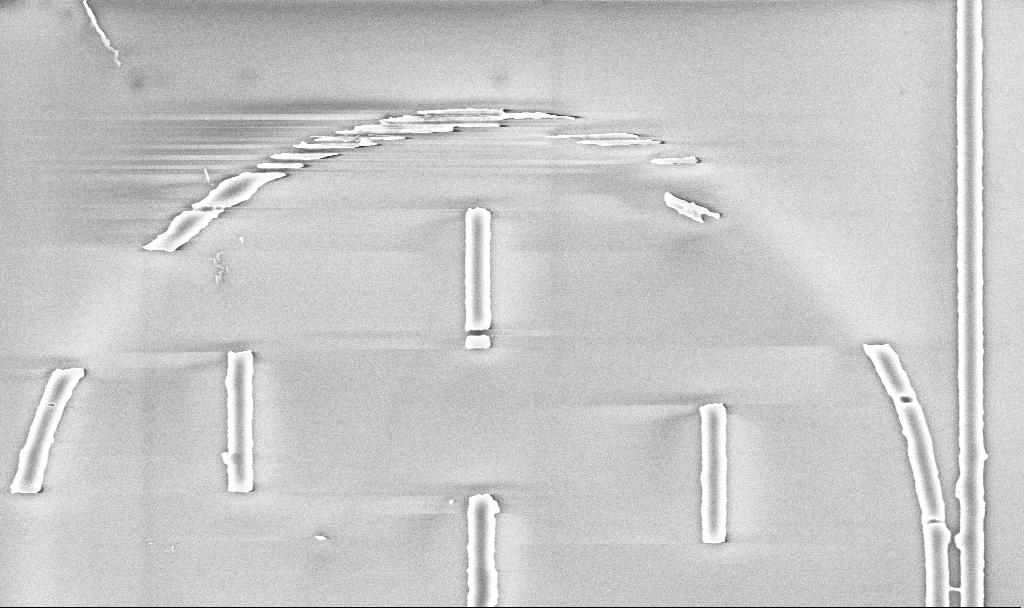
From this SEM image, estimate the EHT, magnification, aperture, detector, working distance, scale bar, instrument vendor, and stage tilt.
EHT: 5 kV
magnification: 17.44 K X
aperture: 30 µm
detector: InLens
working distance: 5.2 mm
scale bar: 2000 nm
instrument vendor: Zeiss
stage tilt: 0°